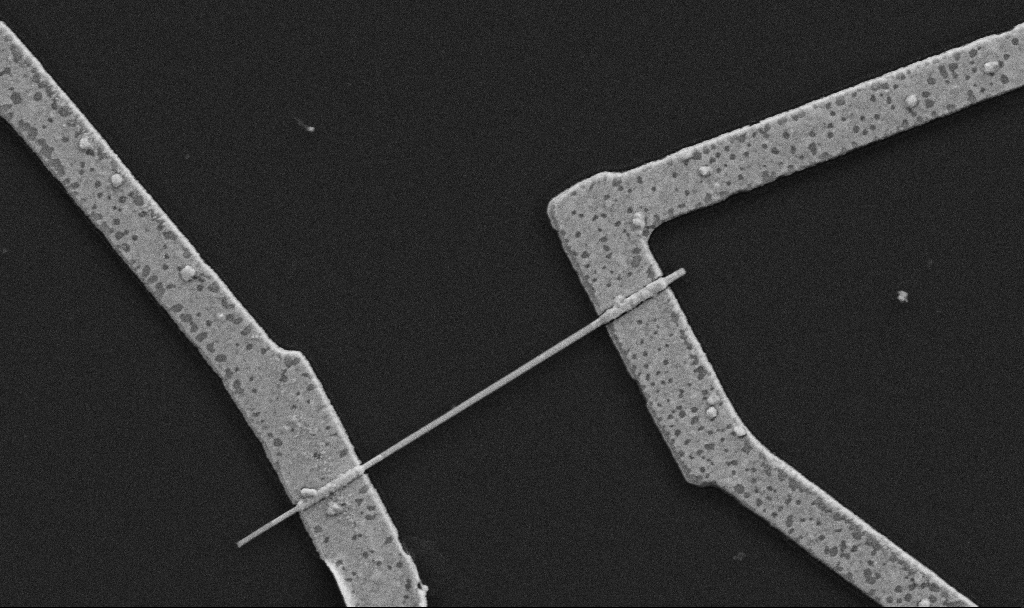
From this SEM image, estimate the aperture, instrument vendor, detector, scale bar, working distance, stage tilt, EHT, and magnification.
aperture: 30 µm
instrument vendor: Zeiss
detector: SE2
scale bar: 1000 nm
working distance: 10.7 mm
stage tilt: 0°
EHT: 5 kV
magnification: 30 K X